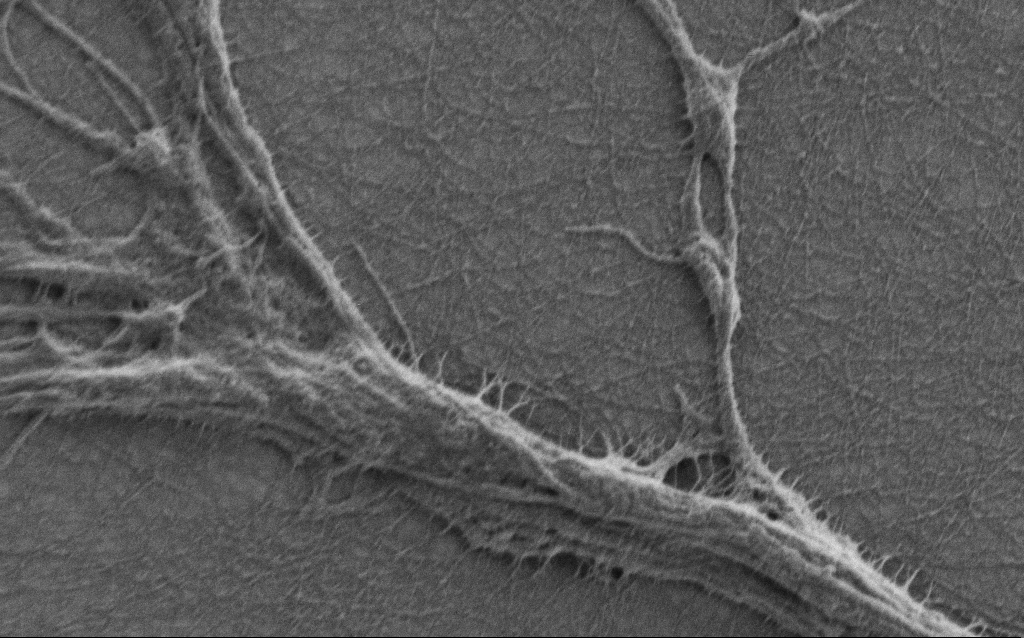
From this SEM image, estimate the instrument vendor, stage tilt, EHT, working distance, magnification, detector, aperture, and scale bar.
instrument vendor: Zeiss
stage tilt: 0°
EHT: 0.9 kV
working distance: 6 mm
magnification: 20 K X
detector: SE2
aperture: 30 µm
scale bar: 2000 nm